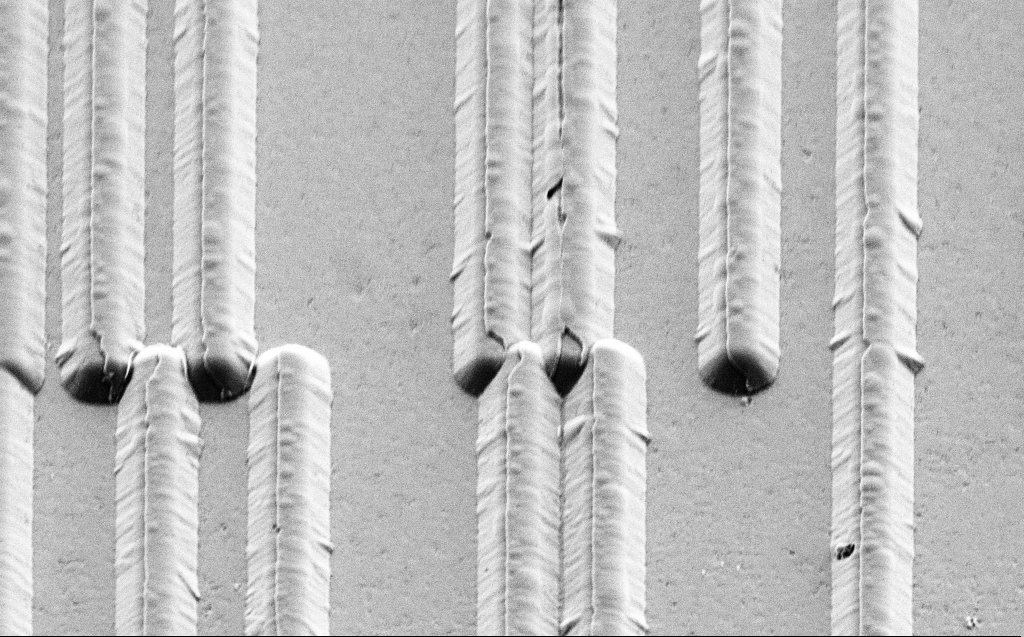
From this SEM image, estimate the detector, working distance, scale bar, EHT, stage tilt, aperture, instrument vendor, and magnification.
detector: SE2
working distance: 11 mm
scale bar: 10000 nm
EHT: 3 kV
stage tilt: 45°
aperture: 30 µm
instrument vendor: Zeiss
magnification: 6.62 K X